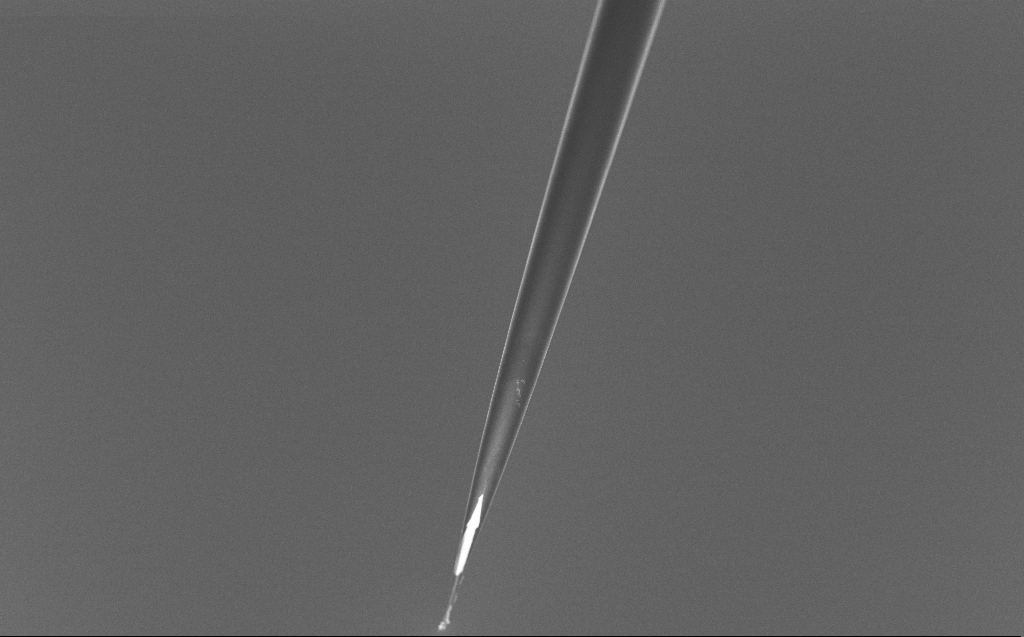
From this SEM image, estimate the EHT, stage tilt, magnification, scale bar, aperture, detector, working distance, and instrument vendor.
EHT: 5 kV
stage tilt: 45°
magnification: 1 K X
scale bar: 20000 nm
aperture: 30 µm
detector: InLens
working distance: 6 mm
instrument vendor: Zeiss